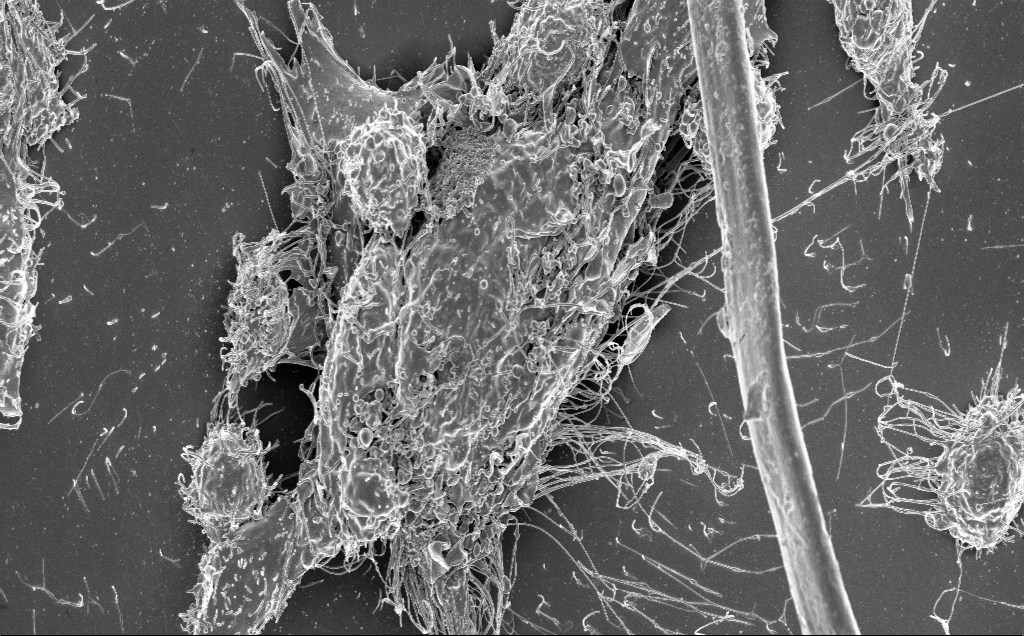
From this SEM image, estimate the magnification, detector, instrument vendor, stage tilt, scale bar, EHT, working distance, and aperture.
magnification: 3.64 K X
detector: InLens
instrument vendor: Zeiss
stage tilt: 0.8°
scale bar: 10000 nm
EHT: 3 kV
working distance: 6 mm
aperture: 30 µm